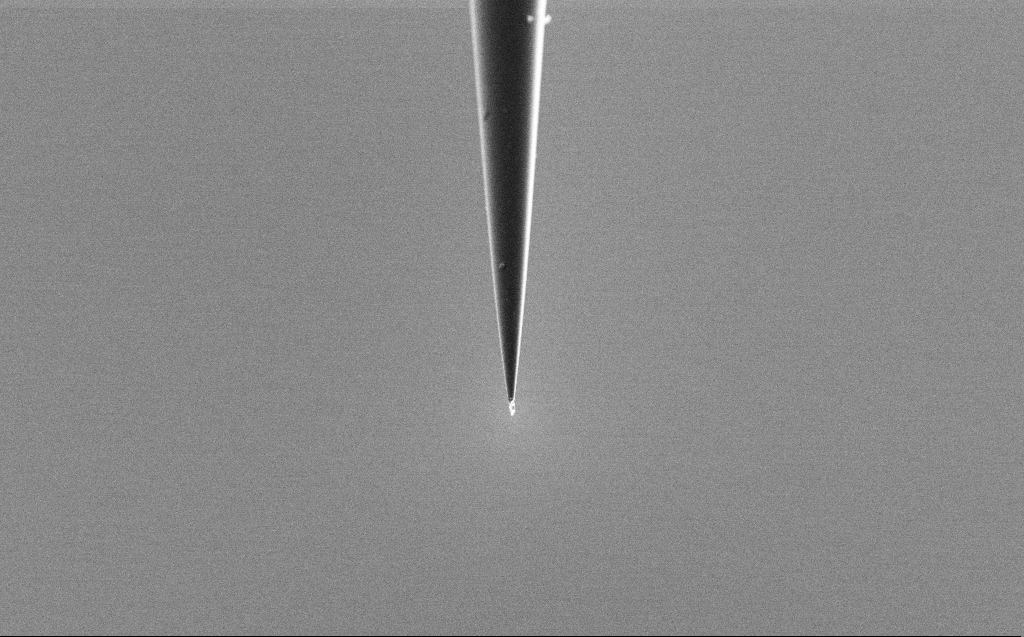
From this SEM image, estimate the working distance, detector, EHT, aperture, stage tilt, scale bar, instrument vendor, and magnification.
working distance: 6 mm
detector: SE2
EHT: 2 kV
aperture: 30 µm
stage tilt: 45°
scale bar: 2000 nm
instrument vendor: Zeiss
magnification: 10 K X